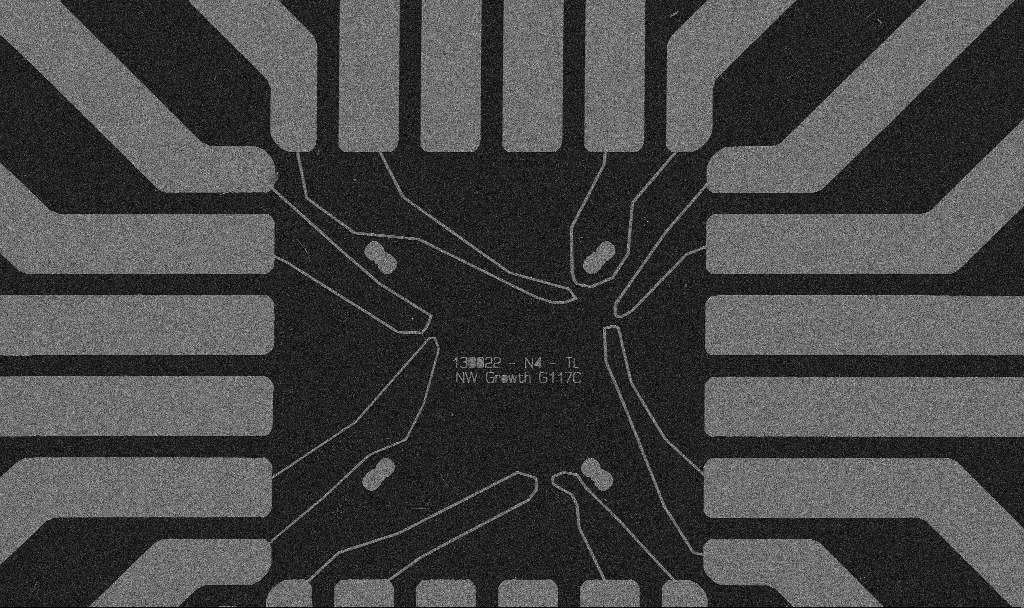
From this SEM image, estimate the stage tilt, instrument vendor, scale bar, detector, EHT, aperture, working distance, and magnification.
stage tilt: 0°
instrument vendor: Zeiss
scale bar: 20000 nm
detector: SE2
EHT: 5 kV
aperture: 30 µm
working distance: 10.7 mm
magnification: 1 K X